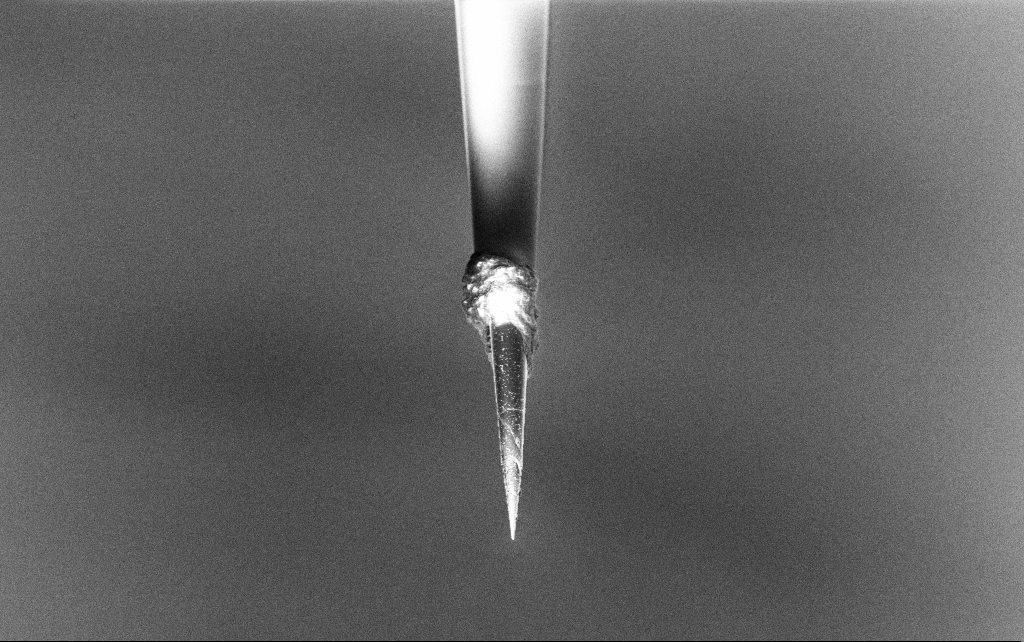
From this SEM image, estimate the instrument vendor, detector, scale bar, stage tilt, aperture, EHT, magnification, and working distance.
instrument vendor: Zeiss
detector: InLens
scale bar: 20000 nm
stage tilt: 45°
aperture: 30 µm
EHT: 3 kV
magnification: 2.5 K X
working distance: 7.7 mm